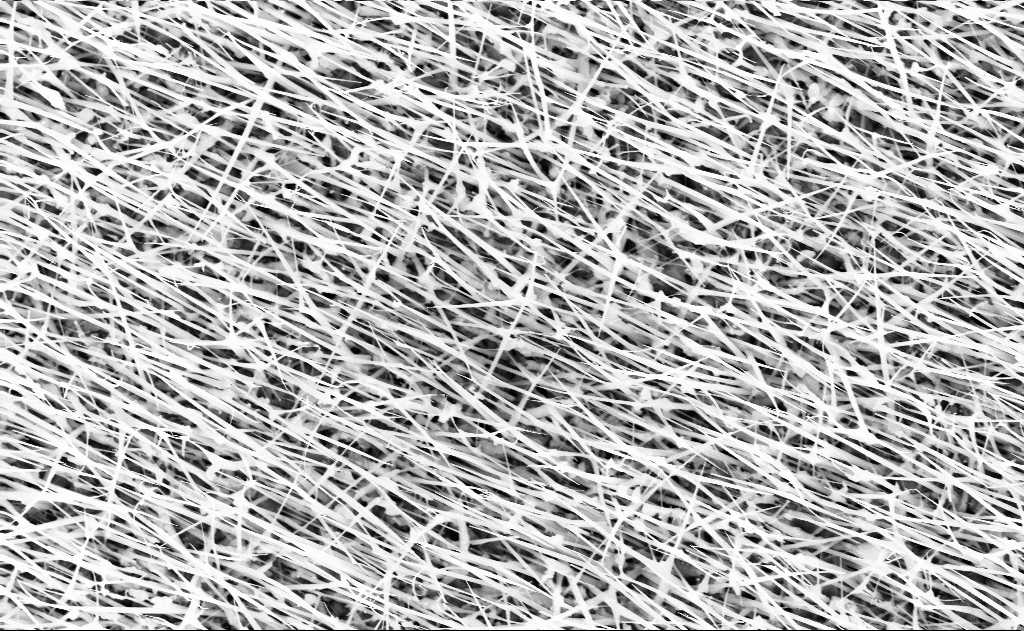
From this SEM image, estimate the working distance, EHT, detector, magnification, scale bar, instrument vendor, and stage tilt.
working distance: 13 mm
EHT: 10 kV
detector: InLens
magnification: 20 K X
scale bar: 2000 nm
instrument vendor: Zeiss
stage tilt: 0°